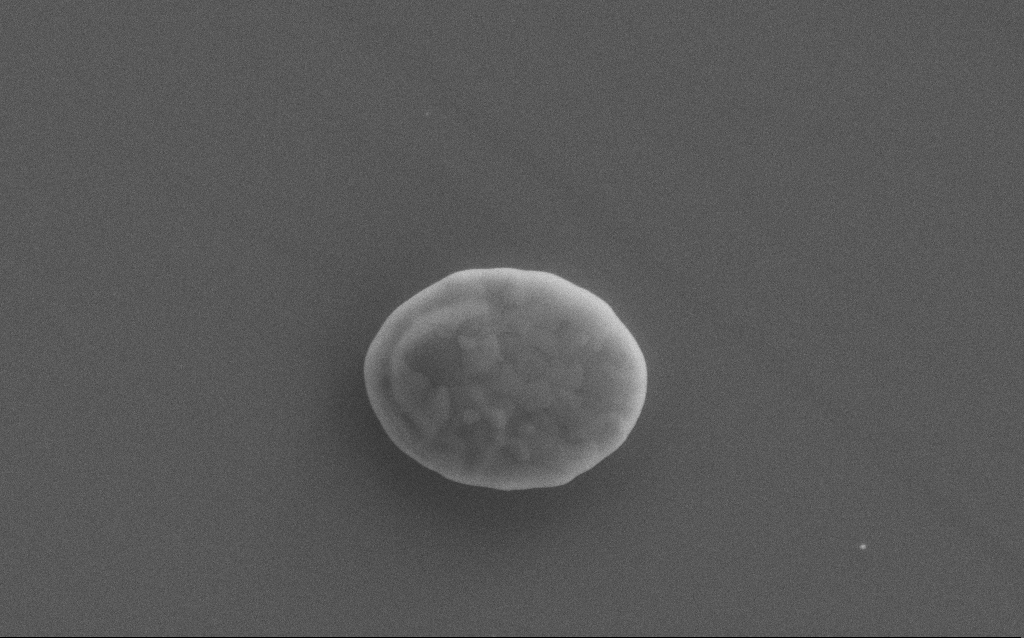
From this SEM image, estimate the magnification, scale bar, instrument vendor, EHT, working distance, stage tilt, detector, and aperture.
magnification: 35.24 K X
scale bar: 2000 nm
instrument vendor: Zeiss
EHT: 10 kV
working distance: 2 mm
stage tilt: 0°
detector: SE2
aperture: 30 µm